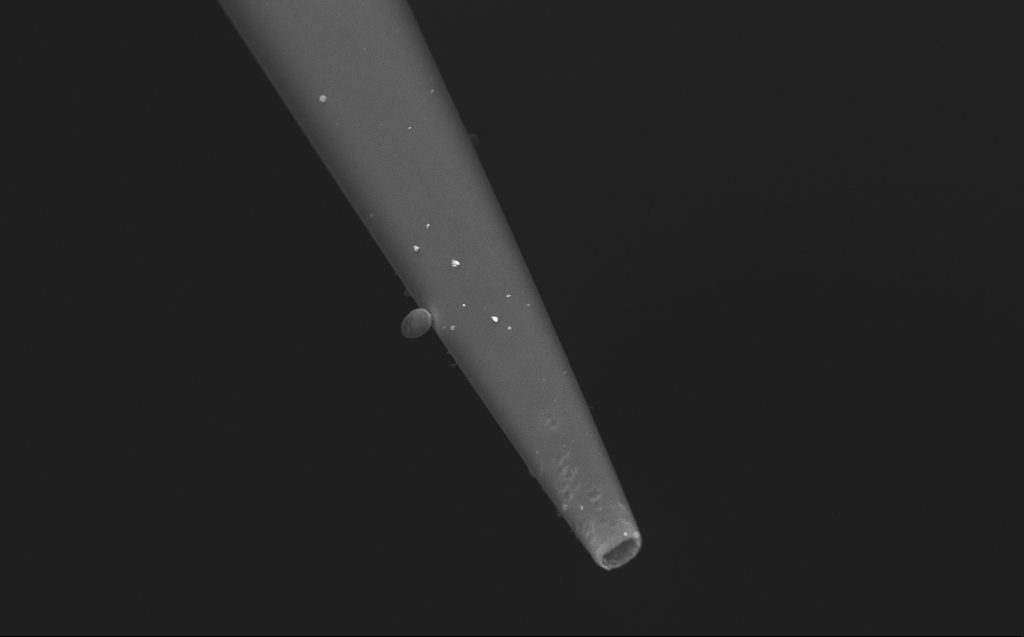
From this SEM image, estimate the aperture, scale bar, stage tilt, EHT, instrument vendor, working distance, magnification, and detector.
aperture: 30 µm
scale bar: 2000 nm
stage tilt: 45°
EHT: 5 kV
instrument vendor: Zeiss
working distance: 4 mm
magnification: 24.02 K X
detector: InLens